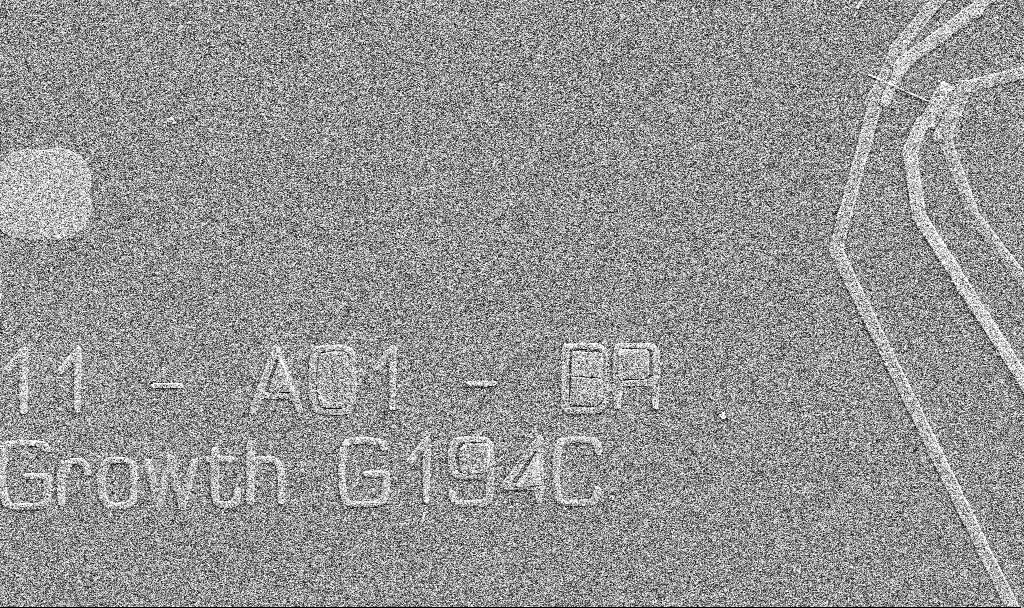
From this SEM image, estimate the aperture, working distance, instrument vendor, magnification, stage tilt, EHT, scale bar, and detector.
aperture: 30 µm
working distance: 10.7 mm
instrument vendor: Zeiss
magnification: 6.29 K X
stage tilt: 0°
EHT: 5 kV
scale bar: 10000 nm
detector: SE2